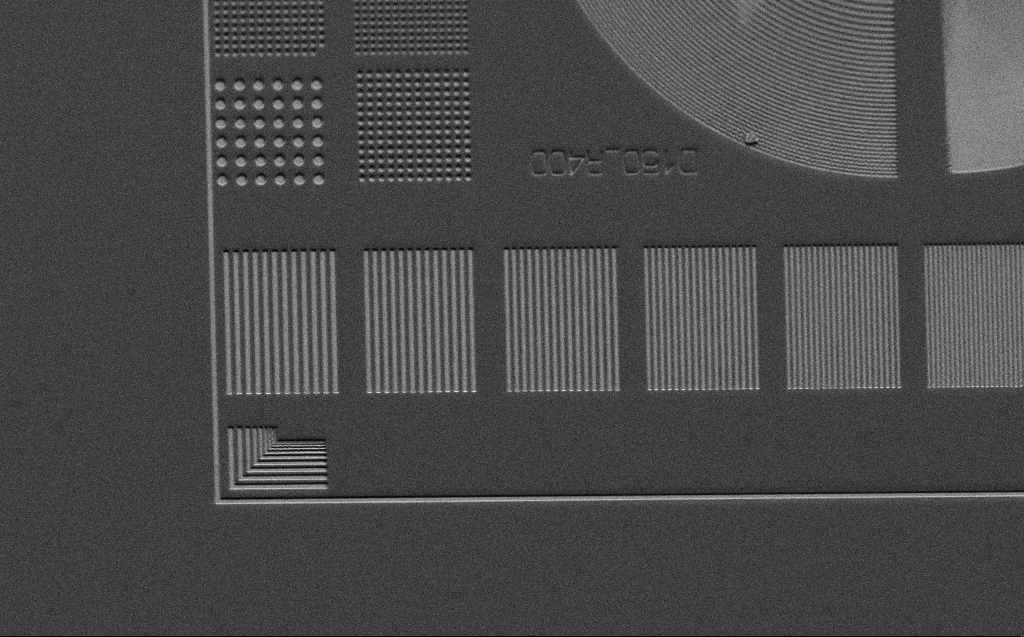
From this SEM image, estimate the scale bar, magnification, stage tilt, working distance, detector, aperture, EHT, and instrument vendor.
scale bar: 10000 nm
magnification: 1.79 K X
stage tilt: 45°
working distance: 6 mm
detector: SE2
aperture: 30 µm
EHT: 3 kV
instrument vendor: Zeiss